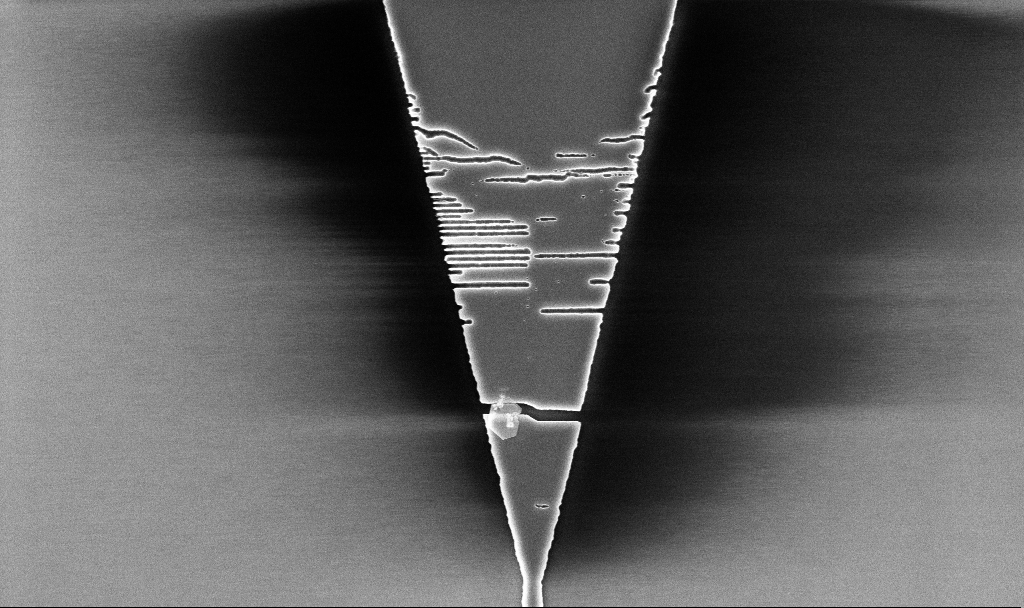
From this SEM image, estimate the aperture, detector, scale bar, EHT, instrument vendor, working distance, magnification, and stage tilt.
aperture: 30 µm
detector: InLens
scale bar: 2000 nm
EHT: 5 kV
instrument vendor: Zeiss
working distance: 5.2 mm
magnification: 14.02 K X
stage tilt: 0°